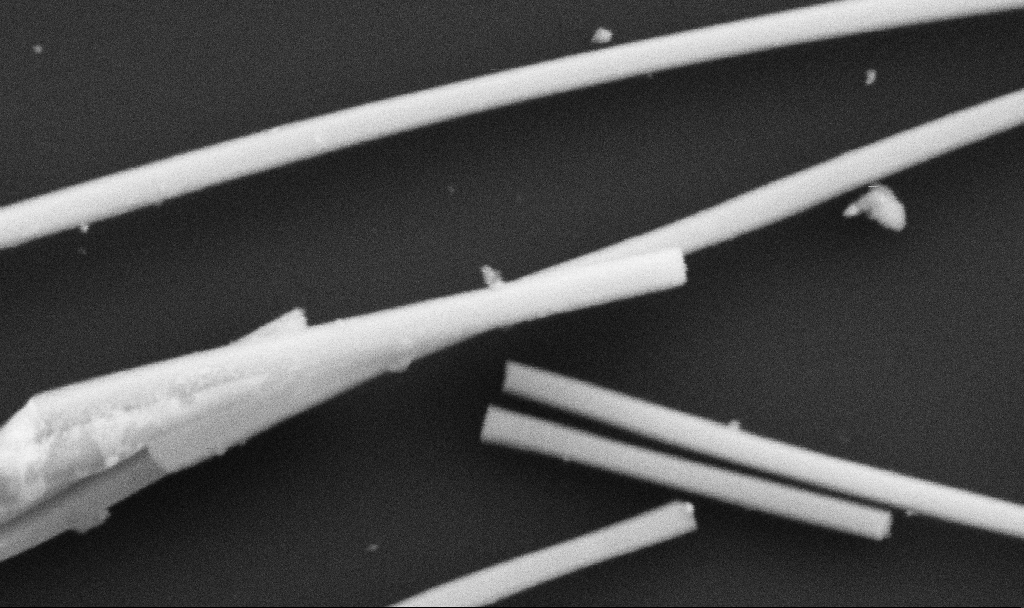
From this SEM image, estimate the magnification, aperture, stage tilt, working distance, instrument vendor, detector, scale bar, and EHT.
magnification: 131.77 K X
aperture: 30 µm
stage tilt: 0°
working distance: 10.7 mm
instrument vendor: Zeiss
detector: SE2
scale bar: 200 nm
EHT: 5 kV